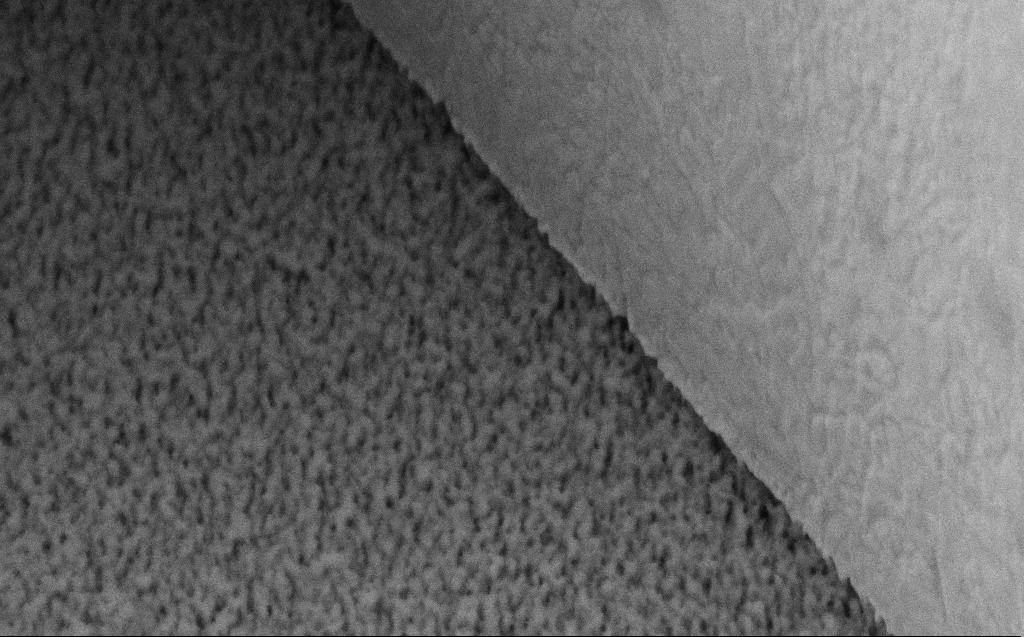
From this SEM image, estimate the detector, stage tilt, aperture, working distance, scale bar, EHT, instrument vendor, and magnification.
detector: SE2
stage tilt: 45°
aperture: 30 µm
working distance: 6 mm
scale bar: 1000 nm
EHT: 5 kV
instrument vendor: Zeiss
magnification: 62.78 K X